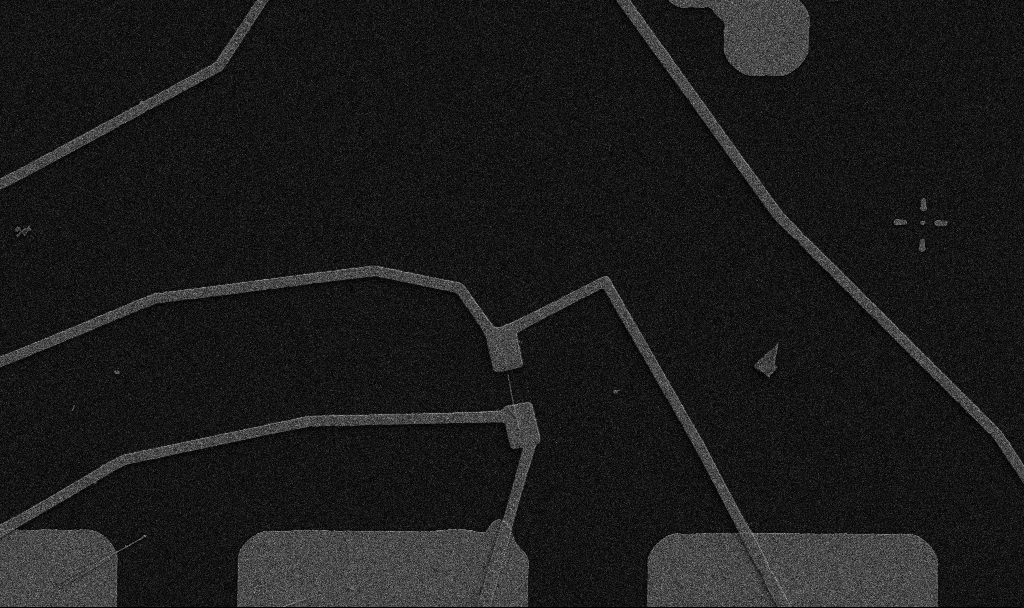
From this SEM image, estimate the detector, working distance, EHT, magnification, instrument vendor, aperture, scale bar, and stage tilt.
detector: SE2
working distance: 10.7 mm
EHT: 5 kV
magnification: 5 K X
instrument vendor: Zeiss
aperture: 30 µm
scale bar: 10000 nm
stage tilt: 0°